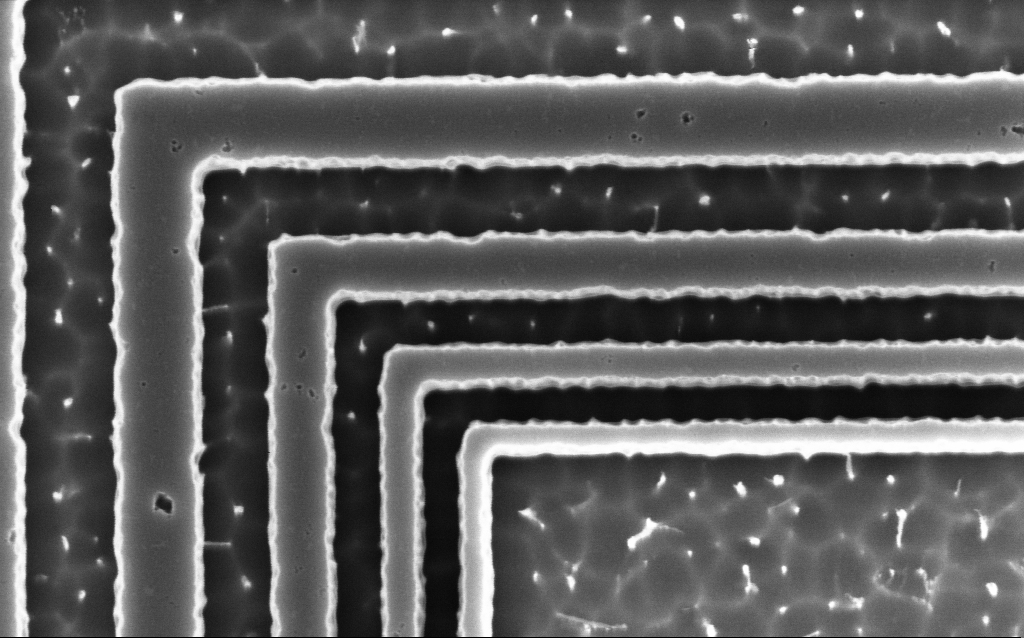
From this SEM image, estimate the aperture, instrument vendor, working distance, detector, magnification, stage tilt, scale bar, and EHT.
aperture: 30 µm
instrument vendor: Zeiss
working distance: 8 mm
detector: InLens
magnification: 80.93 K X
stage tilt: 0°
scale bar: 200 nm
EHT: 3 kV